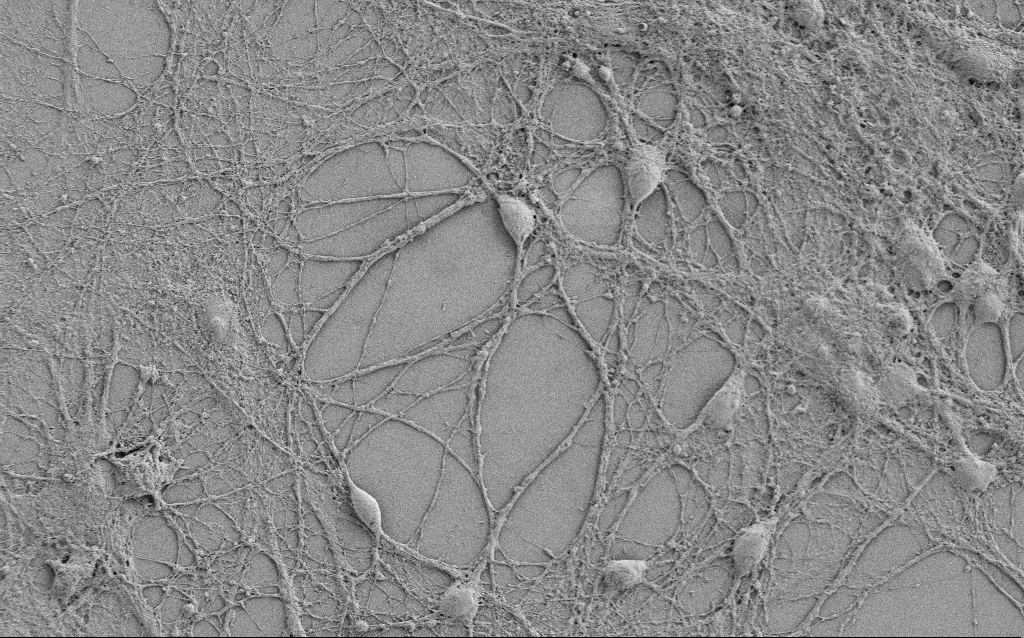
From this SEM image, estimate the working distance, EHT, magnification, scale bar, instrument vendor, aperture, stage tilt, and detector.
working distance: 5.8 mm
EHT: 0.8 kV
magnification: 1.25 K X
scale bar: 20000 nm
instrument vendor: Zeiss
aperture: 30 µm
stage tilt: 0°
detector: SE2